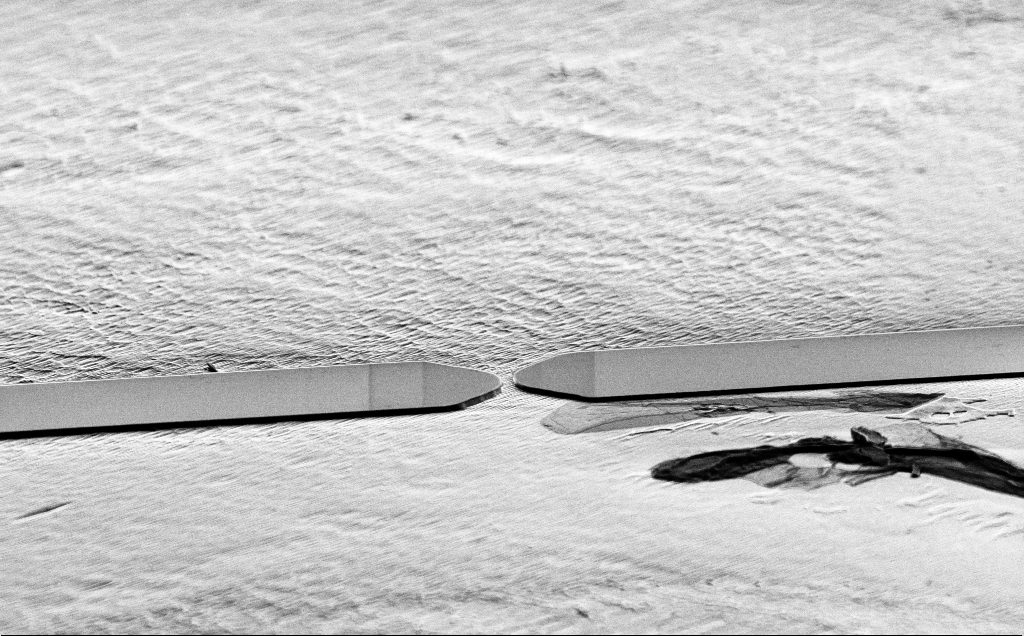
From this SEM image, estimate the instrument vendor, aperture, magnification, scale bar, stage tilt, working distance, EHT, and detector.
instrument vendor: Zeiss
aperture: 30 µm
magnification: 2.68 K X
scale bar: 10000 nm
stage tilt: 70°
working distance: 10 mm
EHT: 5 kV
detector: SE2